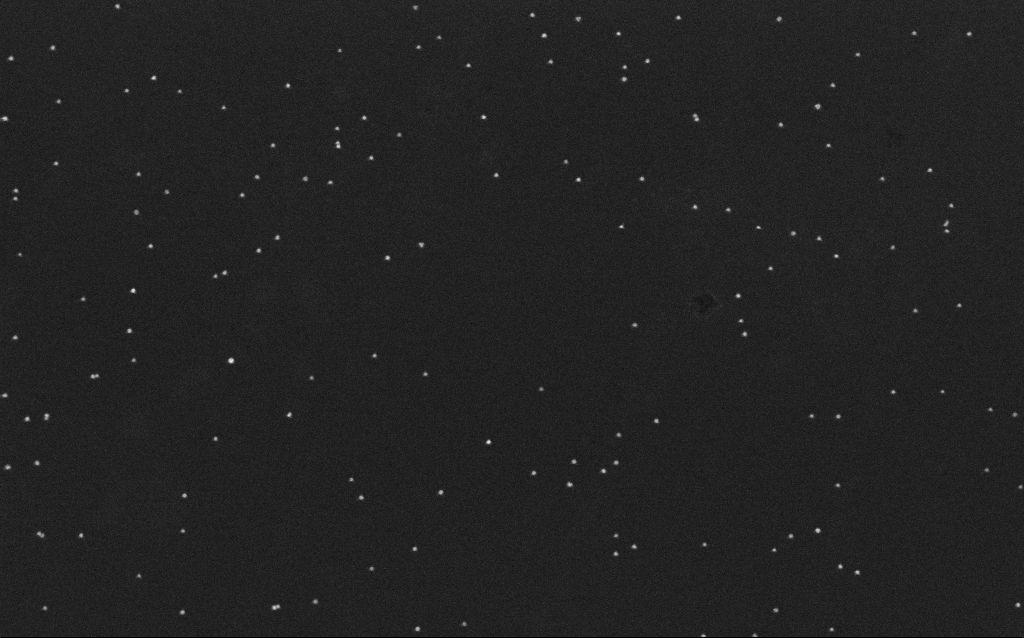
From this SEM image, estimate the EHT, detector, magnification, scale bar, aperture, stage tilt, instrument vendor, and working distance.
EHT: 10 kV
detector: InLens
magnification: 100 K X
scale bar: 200 nm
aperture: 30 µm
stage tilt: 0°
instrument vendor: Zeiss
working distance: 6.5 mm